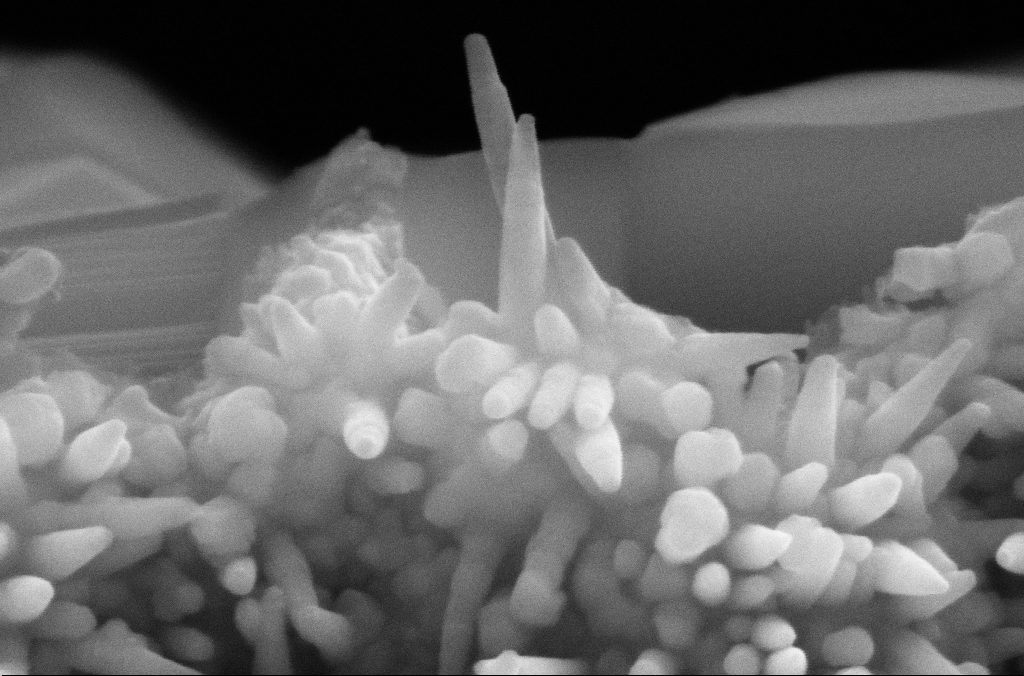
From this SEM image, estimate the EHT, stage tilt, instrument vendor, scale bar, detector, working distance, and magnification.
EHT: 10 kV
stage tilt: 0.1°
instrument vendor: Zeiss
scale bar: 100 nm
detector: SE2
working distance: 5.2 mm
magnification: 279.37 K X